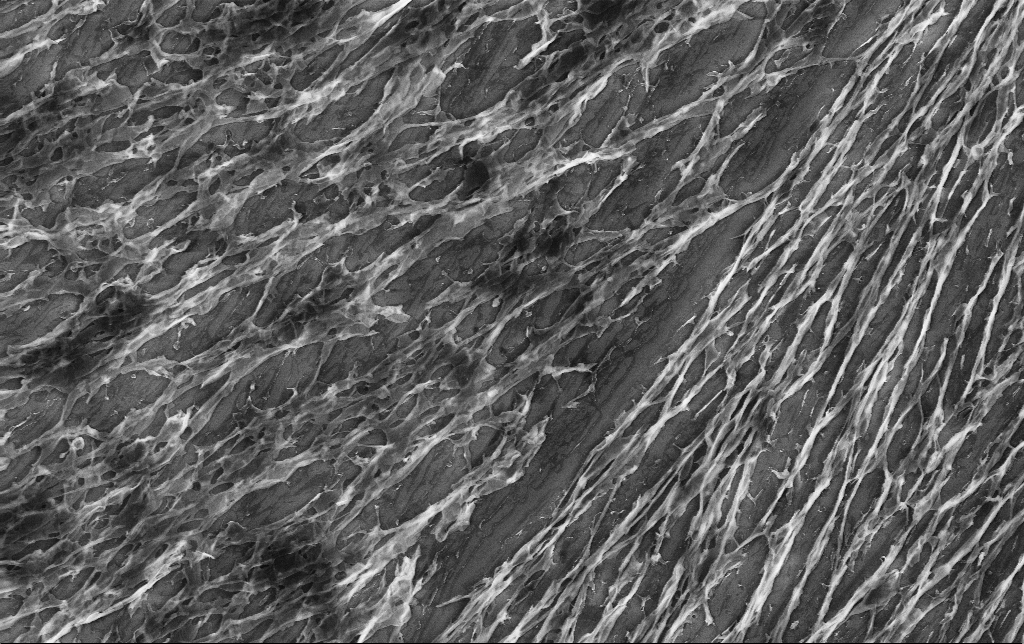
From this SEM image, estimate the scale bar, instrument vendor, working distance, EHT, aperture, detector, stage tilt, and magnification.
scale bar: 10000 nm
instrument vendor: Zeiss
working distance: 3.2 mm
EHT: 10 kV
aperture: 30 µm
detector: InLens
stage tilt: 0°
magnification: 4.41 K X